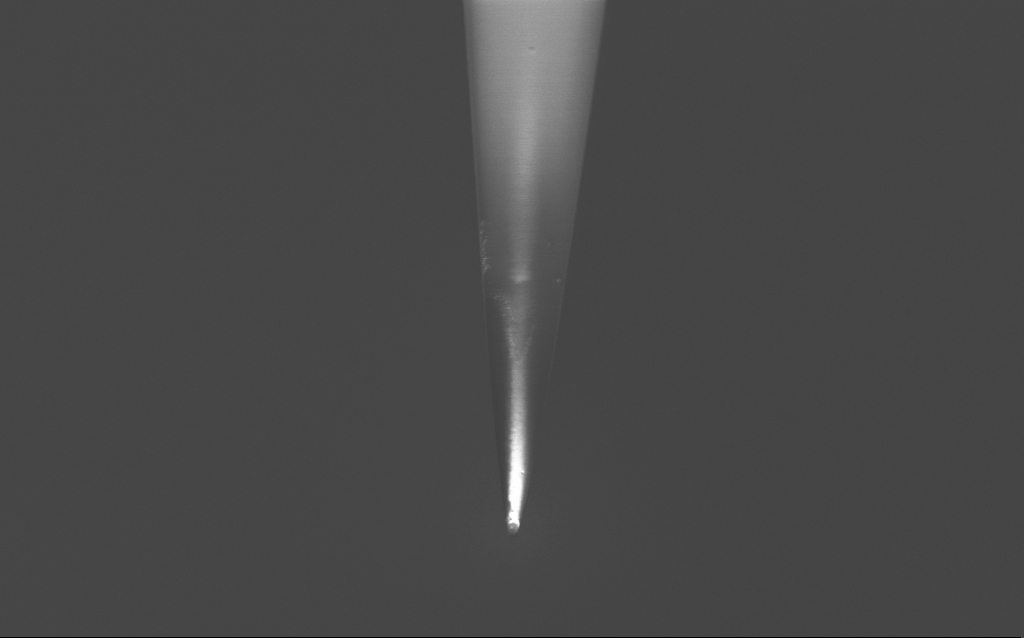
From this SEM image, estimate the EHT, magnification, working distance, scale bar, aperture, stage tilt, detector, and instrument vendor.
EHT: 2 kV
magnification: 10 K X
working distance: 6 mm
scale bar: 2000 nm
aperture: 30 µm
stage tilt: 45°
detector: InLens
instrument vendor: Zeiss